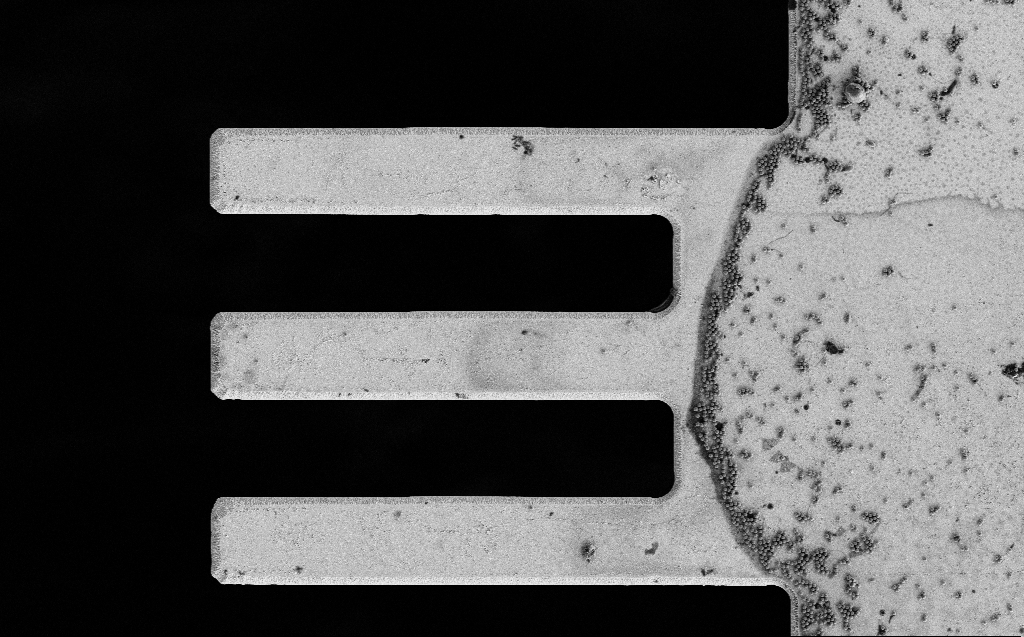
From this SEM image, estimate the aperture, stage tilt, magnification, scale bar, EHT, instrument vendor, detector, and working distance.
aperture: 30 µm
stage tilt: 0°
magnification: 1.71 K X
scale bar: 20000 nm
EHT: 3 kV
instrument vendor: Zeiss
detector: SE2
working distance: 7 mm